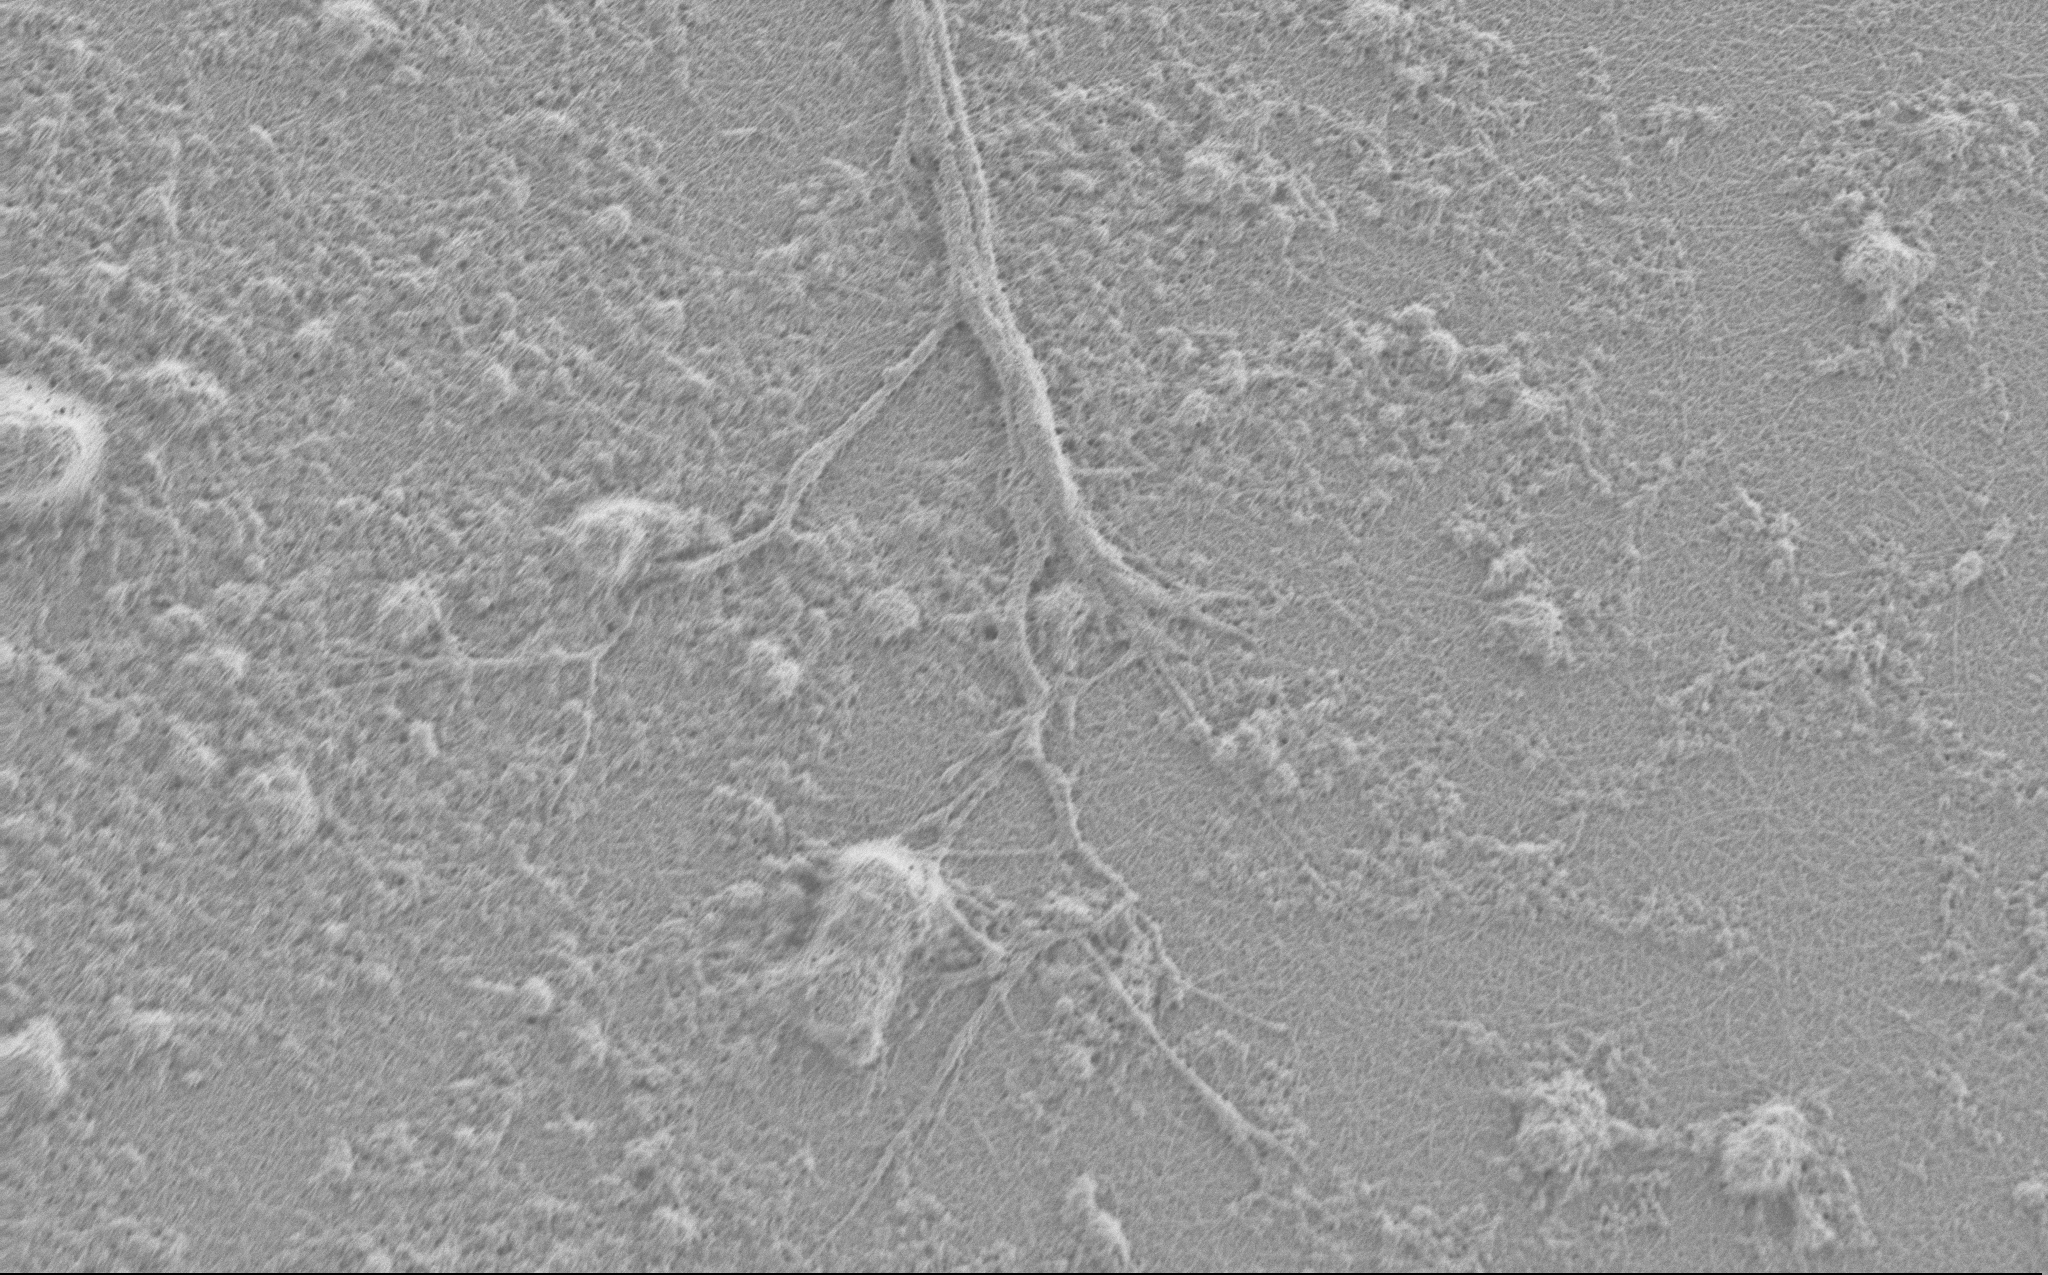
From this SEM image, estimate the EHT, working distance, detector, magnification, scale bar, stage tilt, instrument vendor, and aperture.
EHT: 0.9 kV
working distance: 4 mm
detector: SE2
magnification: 7.5 K X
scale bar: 2000 nm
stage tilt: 0°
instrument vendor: Zeiss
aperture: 30 µm